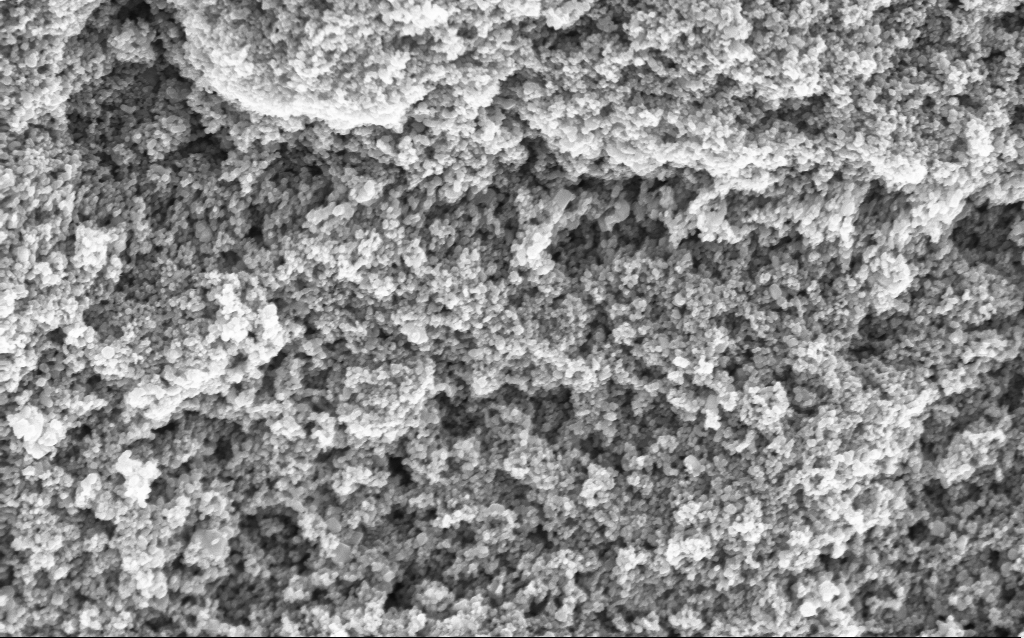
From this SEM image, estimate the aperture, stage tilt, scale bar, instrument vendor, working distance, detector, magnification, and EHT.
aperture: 30 µm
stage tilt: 0°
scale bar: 1000 nm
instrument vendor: Zeiss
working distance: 4.7 mm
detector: InLens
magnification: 68.65 K X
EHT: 5 kV